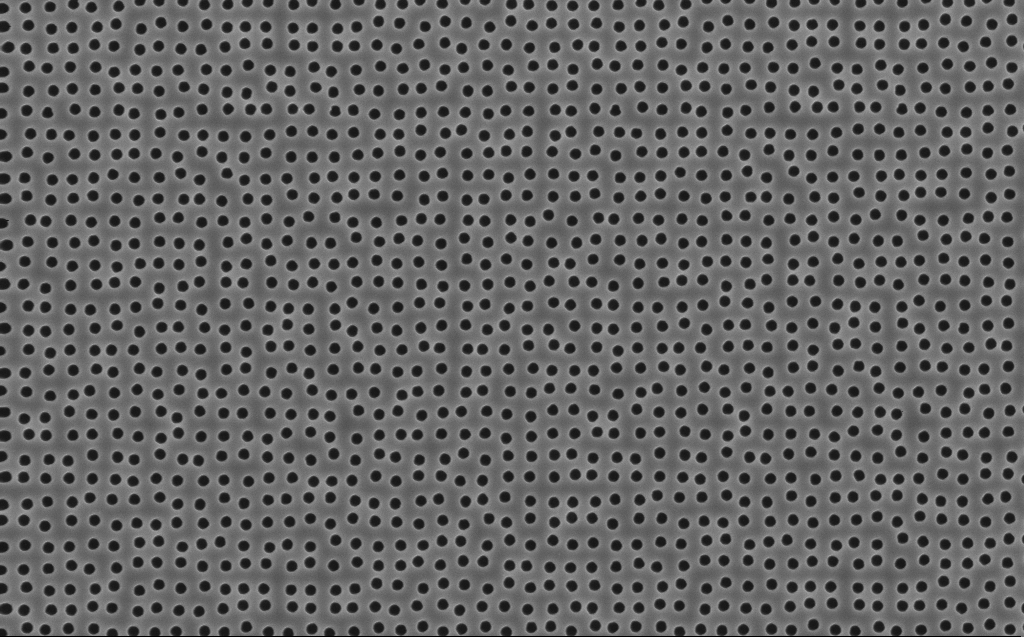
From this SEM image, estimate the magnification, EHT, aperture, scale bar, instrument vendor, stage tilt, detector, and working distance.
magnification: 20.02 K X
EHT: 5 kV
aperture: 30 µm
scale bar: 2000 nm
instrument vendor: Zeiss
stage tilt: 0°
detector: InLens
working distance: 7 mm